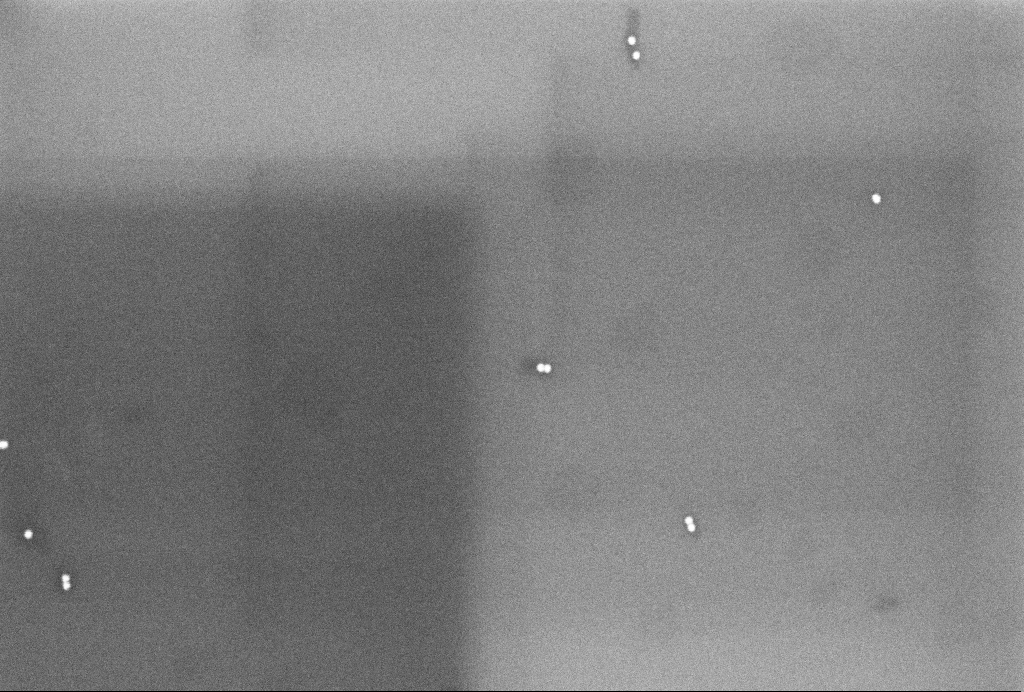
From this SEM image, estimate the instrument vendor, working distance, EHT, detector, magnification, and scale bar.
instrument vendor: Zeiss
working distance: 3.2 mm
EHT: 3 kV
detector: InLens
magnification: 102.77 K X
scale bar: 200 nm